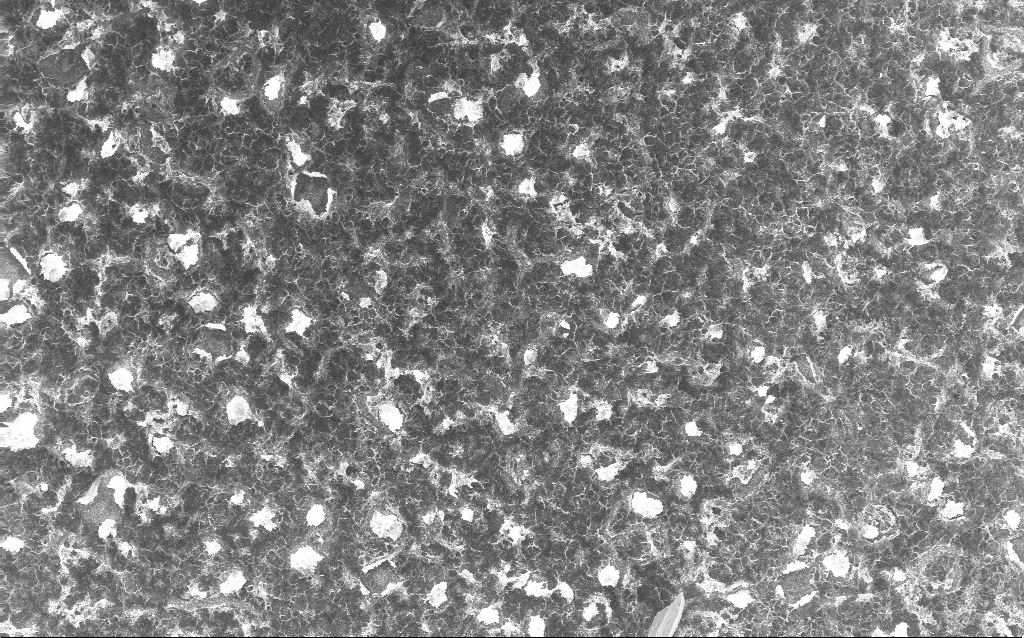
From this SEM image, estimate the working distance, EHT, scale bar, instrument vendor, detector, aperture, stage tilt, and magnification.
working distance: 2.8 mm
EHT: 10 kV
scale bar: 20000 nm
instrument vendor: Zeiss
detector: InLens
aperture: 30 µm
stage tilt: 0°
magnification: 1 K X